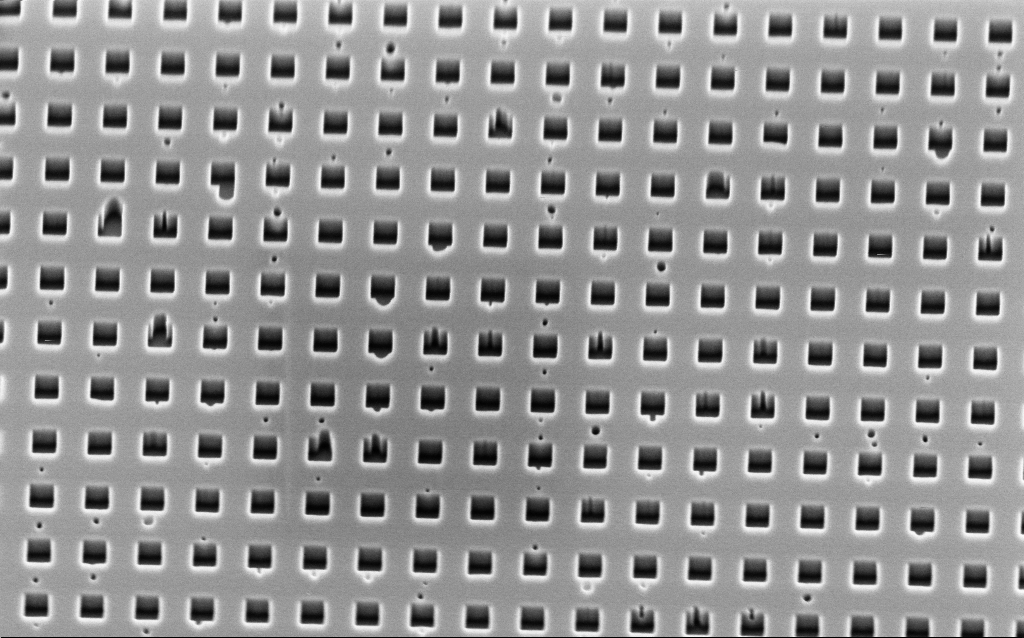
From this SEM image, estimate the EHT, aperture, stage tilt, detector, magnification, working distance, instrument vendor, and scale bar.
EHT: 2 kV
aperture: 30 µm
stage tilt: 45°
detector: InLens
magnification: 40.68 K X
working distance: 2.7 mm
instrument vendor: Zeiss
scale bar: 1000 nm